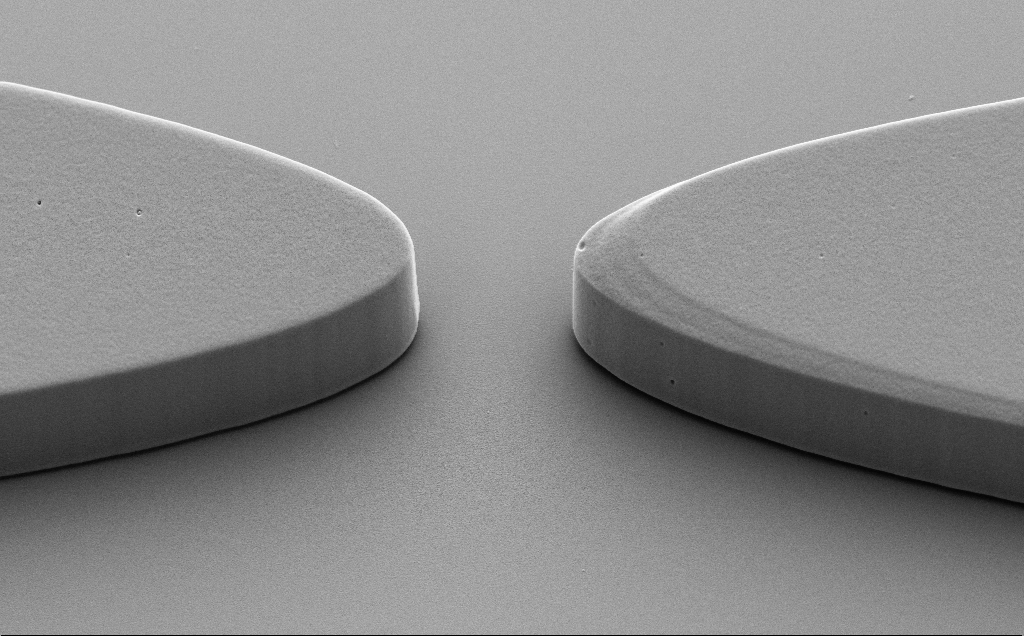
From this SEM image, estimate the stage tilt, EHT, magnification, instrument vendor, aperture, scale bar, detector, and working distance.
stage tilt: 40°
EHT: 5 kV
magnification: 5 K X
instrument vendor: Zeiss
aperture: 30 µm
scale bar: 10000 nm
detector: SE2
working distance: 9 mm